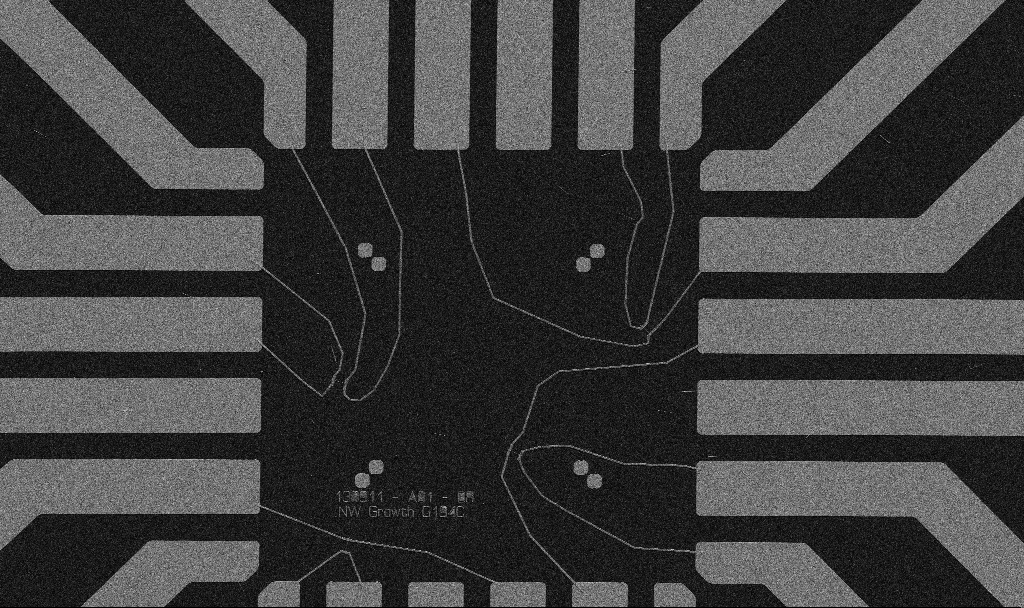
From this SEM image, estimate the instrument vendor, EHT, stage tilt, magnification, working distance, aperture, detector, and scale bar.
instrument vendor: Zeiss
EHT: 5 kV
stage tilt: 0°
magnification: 1 K X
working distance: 10.7 mm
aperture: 30 µm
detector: SE2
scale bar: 20000 nm